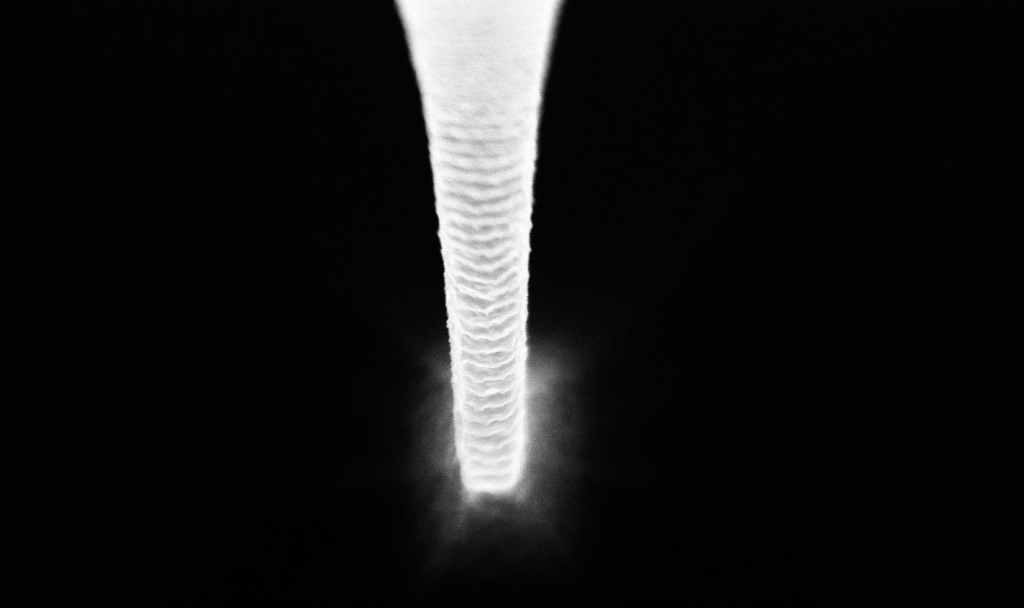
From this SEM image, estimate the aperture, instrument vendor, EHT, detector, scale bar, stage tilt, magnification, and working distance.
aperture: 30 µm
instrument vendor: Zeiss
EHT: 5 kV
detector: InLens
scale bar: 1000 nm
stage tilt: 15°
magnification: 55.41 K X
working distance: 4.8 mm